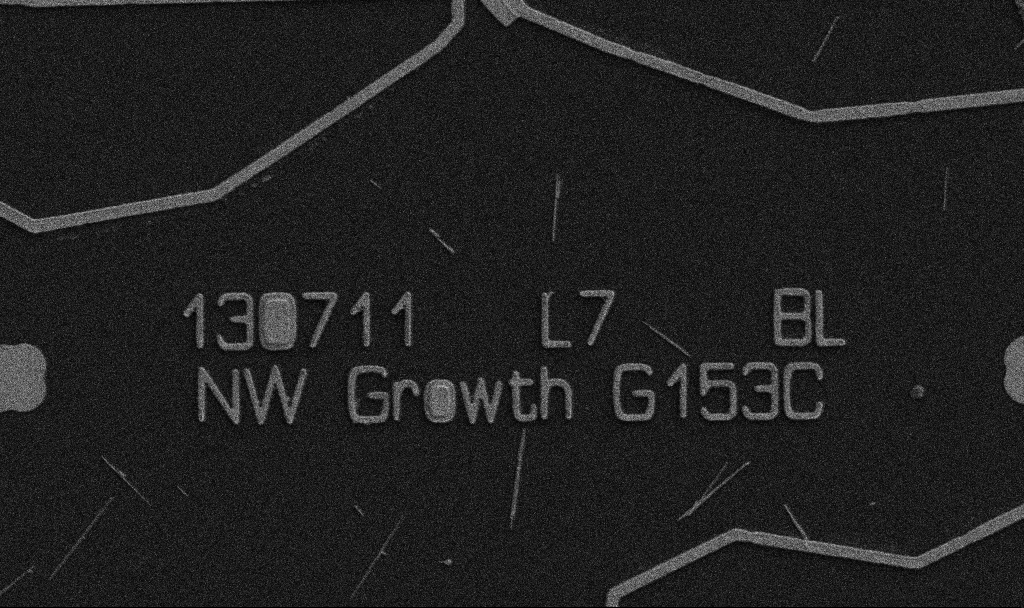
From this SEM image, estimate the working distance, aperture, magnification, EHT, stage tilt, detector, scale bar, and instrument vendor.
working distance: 10.7 mm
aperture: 30 µm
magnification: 5 K X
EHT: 5 kV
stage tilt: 0°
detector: SE2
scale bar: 10000 nm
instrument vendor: Zeiss